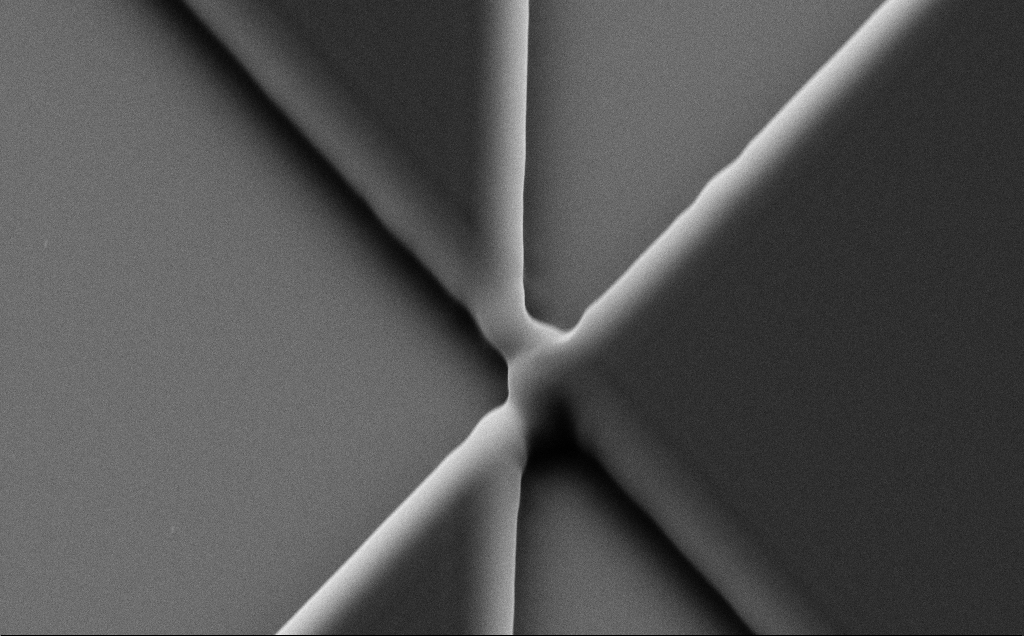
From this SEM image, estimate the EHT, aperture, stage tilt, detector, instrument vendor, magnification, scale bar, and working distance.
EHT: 10 kV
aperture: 30 µm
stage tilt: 35°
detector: SE2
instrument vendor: Zeiss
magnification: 11.83 K X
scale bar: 2000 nm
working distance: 8 mm